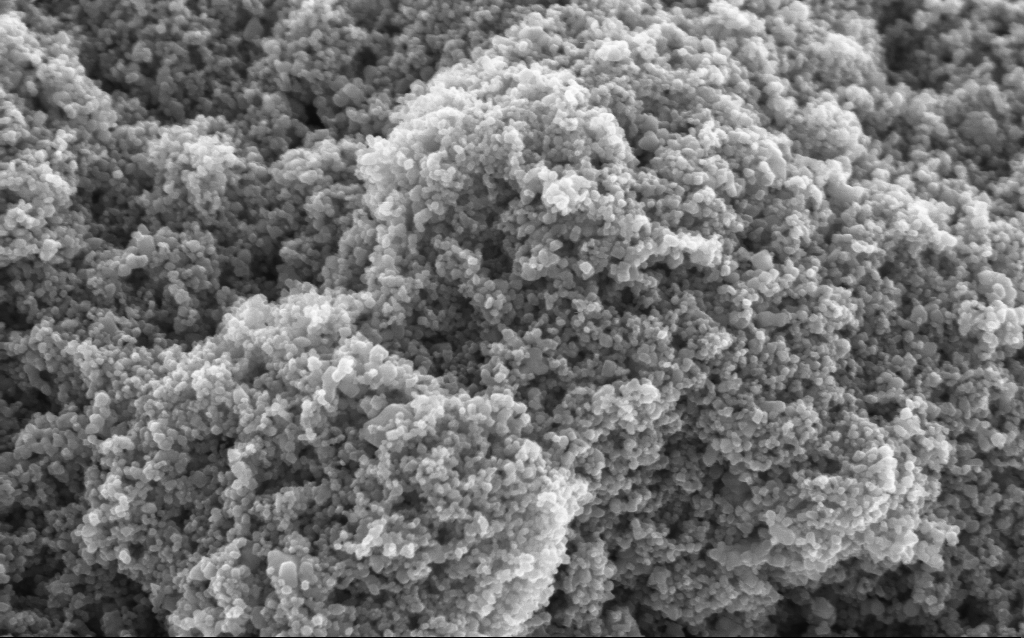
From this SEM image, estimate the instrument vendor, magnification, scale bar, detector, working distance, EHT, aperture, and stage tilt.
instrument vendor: Zeiss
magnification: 114.55 K X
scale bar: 200 nm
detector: InLens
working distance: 4 mm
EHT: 5 kV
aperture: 30 µm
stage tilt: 0°